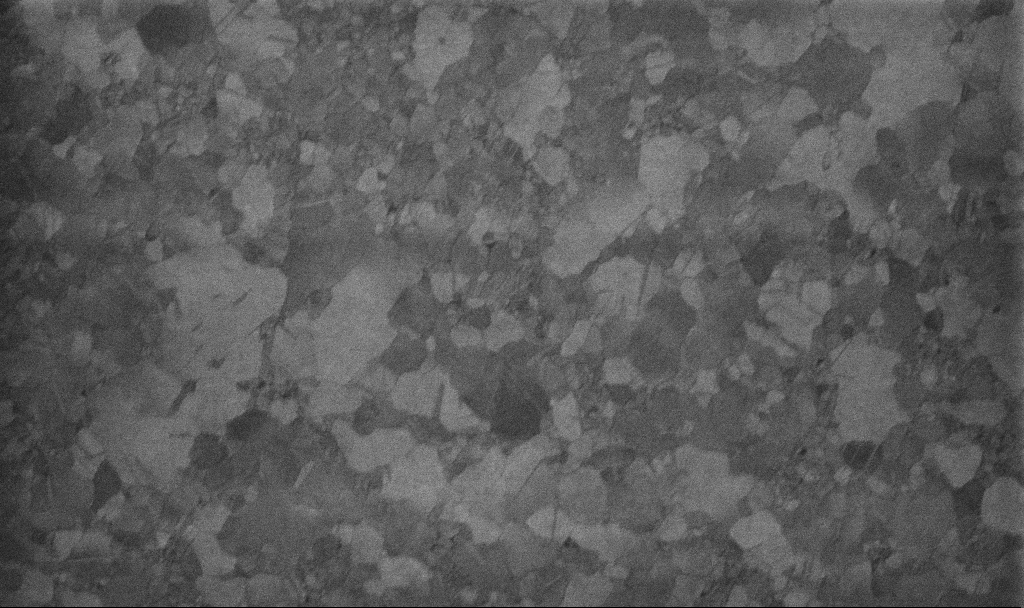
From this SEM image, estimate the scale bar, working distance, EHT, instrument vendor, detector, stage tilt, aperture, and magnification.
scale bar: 200 nm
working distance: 3.4 mm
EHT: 10 kV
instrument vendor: Zeiss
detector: InLens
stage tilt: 0°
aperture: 30 µm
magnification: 100 K X